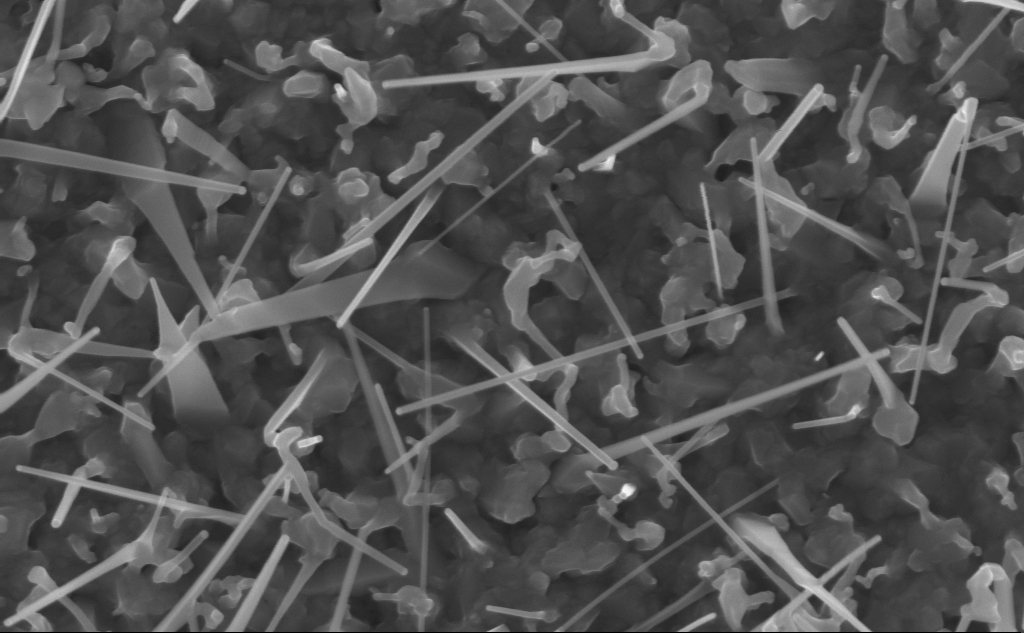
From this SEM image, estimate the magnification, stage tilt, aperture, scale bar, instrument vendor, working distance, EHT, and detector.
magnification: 80 K X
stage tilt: -0°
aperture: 30 µm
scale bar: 200 nm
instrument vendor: Zeiss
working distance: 5 mm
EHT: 10 kV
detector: InLens